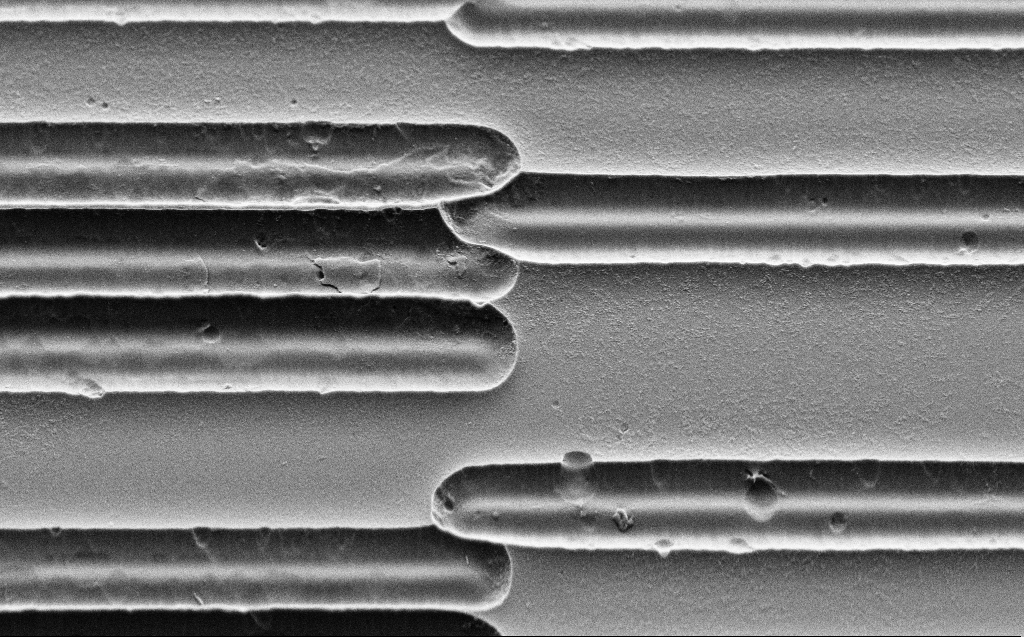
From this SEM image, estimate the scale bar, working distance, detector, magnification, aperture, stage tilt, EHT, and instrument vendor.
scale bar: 10000 nm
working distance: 6 mm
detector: SE2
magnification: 6.96 K X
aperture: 30 µm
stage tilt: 45°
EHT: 3 kV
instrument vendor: Zeiss